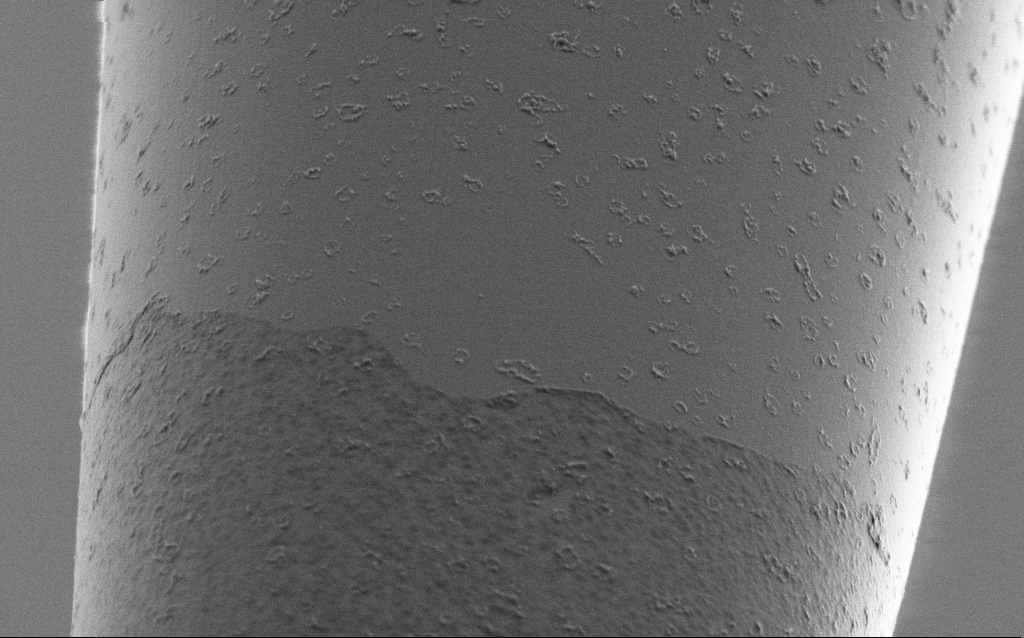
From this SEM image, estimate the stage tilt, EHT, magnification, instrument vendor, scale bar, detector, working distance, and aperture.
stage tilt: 45°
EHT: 1 kV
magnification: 25 K X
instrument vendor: Zeiss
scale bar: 1000 nm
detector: SE2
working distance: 6 mm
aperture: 30 µm